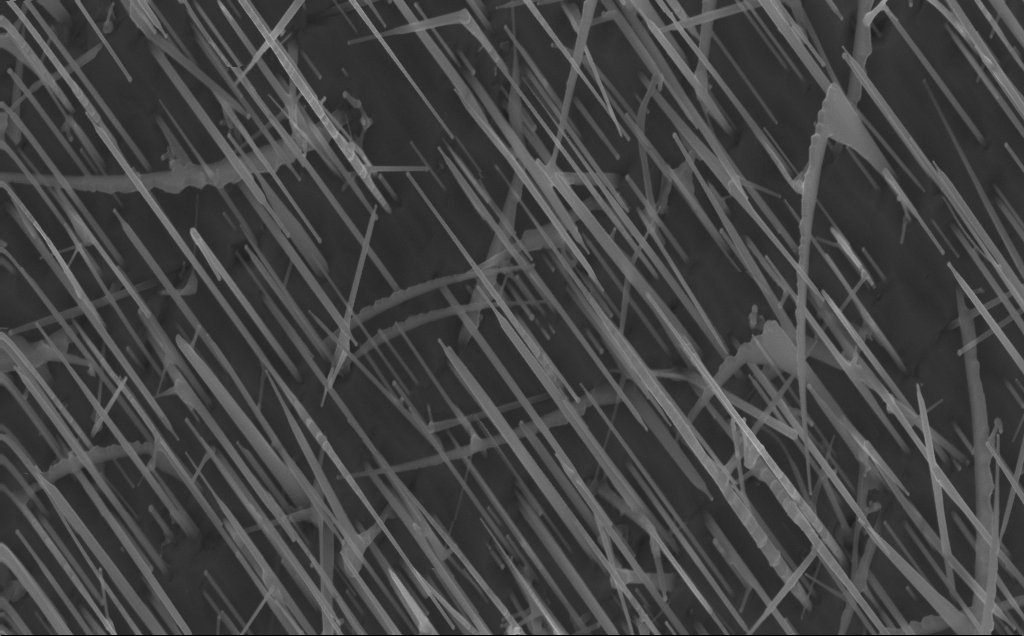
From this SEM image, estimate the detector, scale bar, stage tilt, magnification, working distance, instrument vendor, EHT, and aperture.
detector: InLens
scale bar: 1000 nm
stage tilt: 0°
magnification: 40 K X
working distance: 4 mm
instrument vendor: Zeiss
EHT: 10 kV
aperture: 30 µm